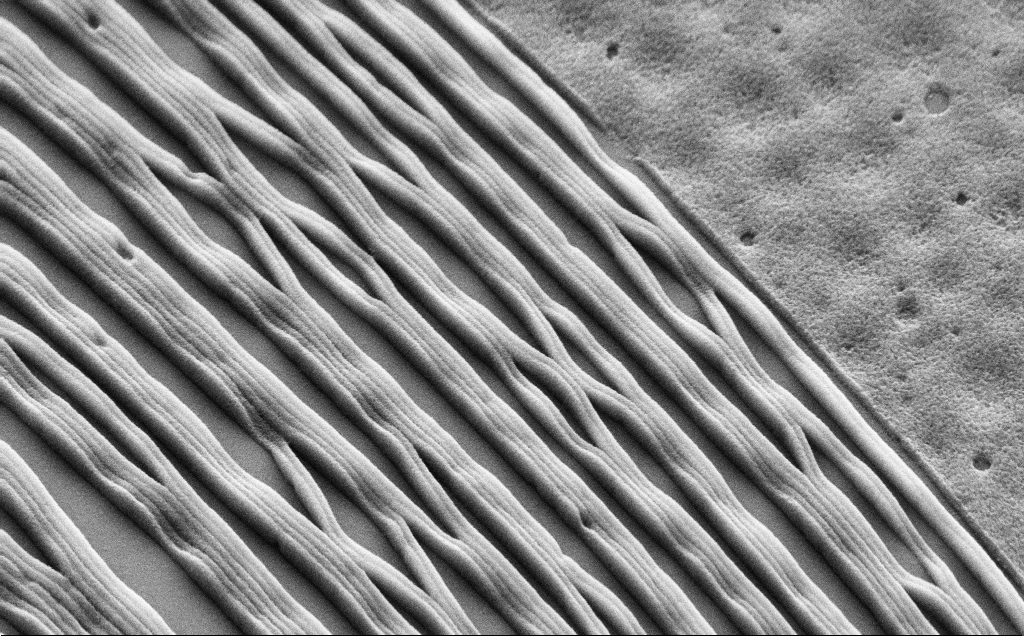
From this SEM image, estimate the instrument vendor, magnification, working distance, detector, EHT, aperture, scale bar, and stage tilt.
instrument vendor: Zeiss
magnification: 32.47 K X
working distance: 6 mm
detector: SE2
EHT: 5 kV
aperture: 30 µm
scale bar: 2000 nm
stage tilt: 45°